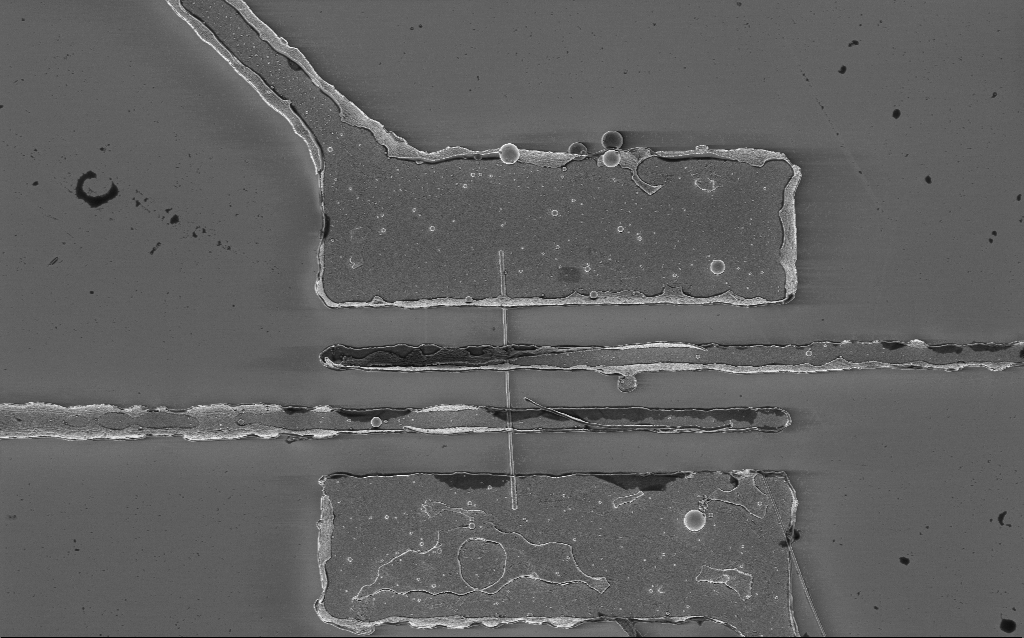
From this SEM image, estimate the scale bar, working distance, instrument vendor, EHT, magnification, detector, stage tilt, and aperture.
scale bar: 10000 nm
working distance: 8.1 mm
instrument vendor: Zeiss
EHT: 2 kV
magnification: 5.85 K X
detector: InLens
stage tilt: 0°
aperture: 30 µm